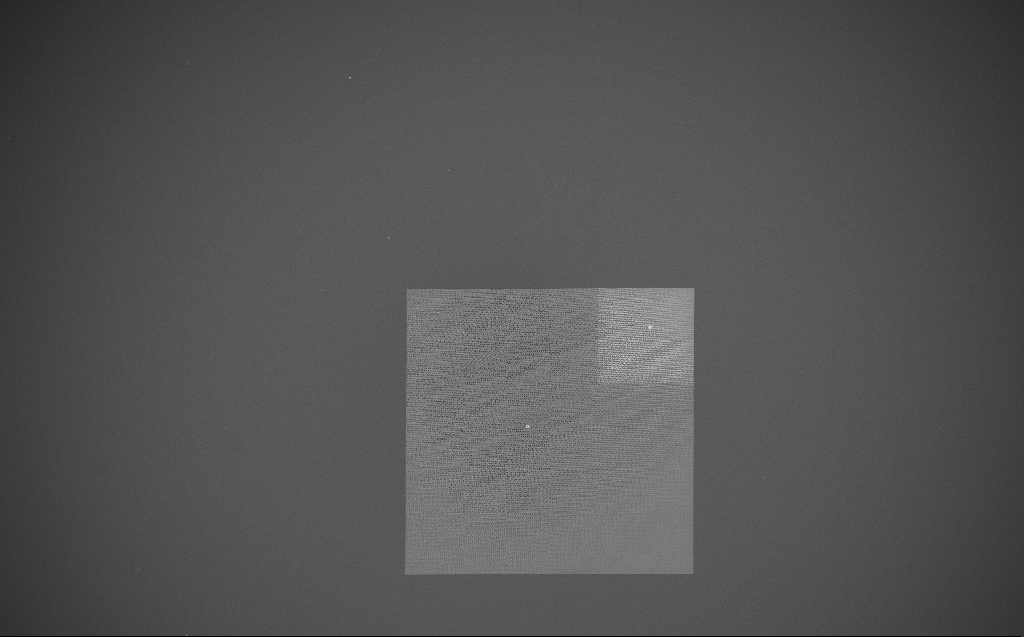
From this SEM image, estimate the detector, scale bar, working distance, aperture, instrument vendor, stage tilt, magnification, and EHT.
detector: InLens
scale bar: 100000 nm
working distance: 7 mm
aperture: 30 µm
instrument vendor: Zeiss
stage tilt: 0°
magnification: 0.143 K X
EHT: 5 kV